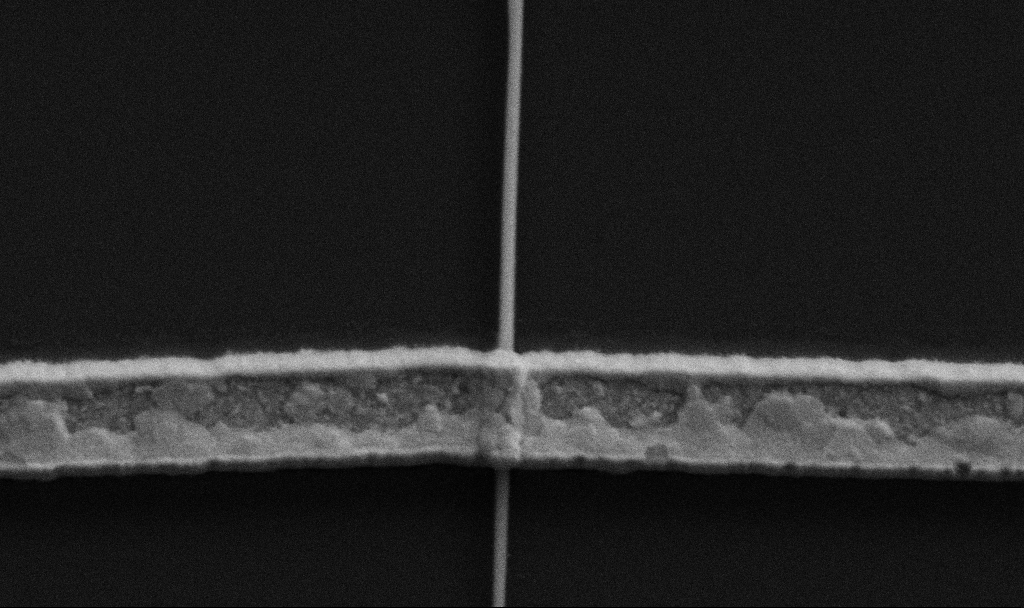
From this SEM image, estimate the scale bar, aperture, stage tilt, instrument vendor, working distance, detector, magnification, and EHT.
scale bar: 1000 nm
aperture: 30 µm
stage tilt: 0°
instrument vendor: Zeiss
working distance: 8.5 mm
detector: SE2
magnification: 60 K X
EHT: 5 kV